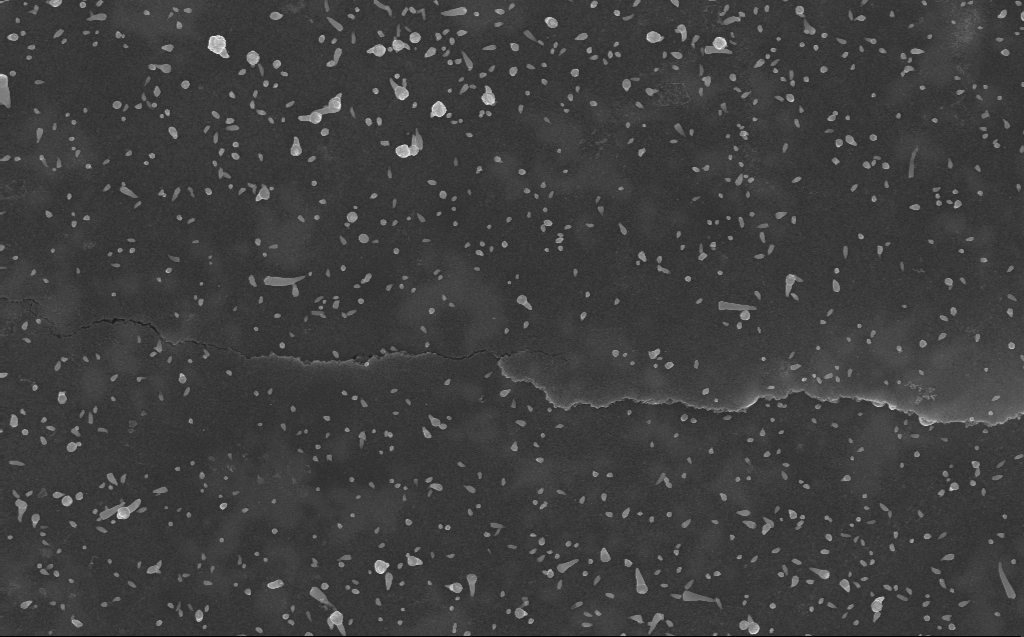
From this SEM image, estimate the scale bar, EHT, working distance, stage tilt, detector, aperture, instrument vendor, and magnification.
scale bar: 1000 nm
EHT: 10 kV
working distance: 3 mm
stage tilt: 0°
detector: InLens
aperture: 30 µm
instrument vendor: Zeiss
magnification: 18.88 K X